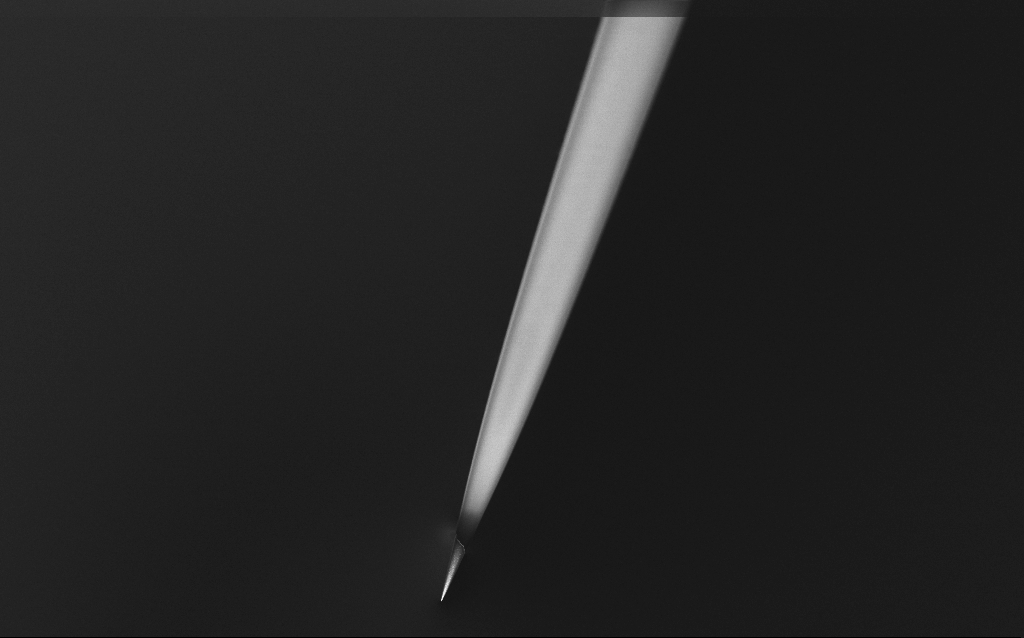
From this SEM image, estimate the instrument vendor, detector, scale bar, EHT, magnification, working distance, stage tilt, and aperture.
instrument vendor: Zeiss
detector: InLens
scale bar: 20000 nm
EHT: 1 kV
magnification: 1 K X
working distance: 6 mm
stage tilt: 45°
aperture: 30 µm